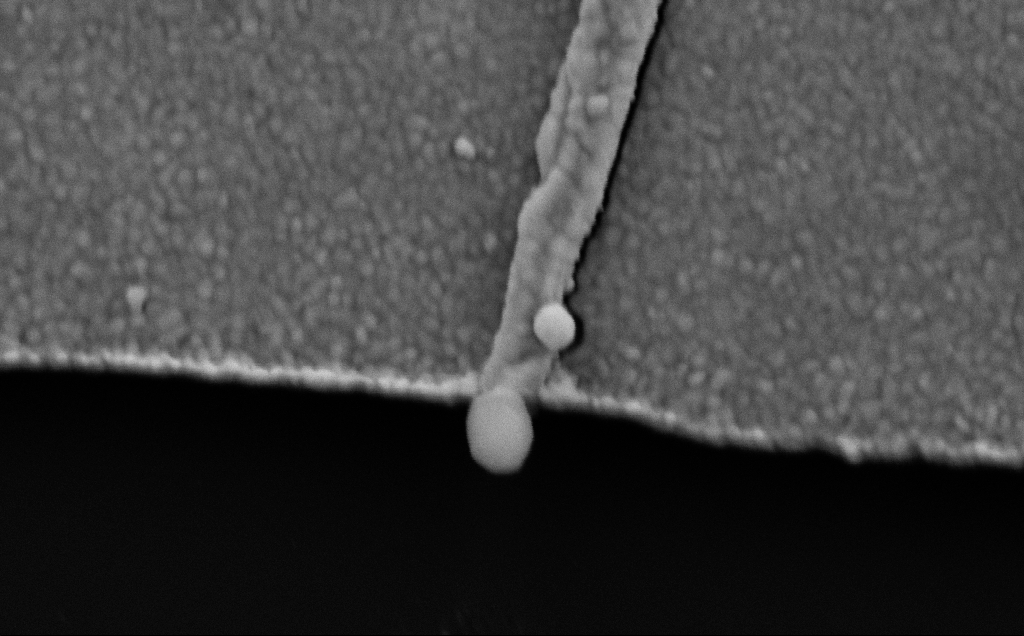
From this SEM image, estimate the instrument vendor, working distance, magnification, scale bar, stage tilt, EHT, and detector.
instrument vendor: Zeiss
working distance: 8 mm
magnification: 145.91 K X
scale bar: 200 nm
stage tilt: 0°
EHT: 5 kV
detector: SE2